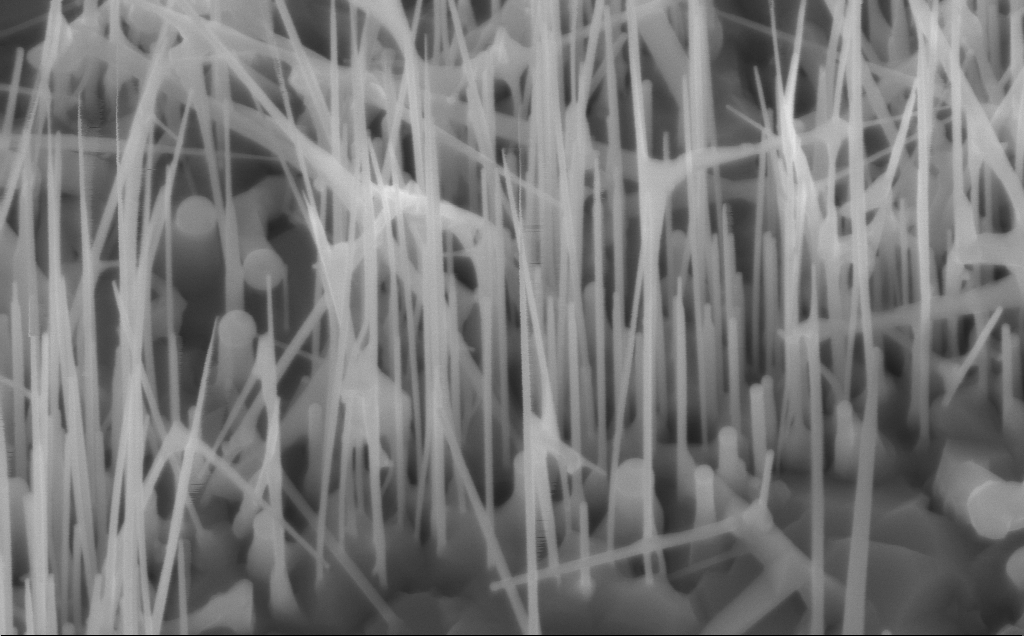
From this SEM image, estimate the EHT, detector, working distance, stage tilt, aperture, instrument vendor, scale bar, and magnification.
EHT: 10 kV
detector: InLens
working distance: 5 mm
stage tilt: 30°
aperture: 30 µm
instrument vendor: Zeiss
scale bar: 200 nm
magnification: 80 K X